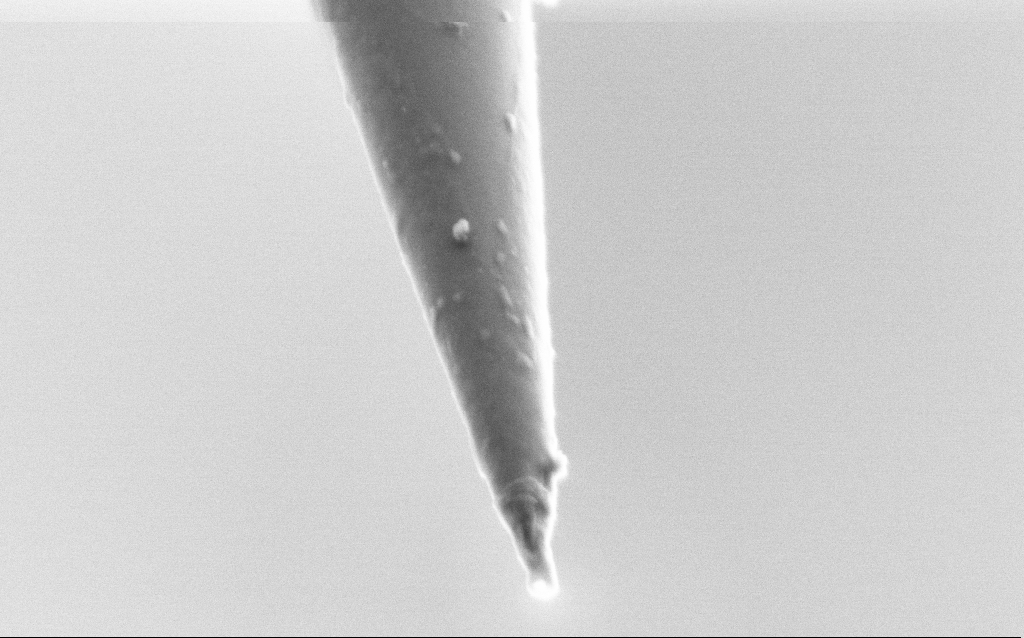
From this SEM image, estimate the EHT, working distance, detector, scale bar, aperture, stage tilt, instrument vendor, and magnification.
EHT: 1.5 kV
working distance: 6 mm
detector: SE2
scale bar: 200 nm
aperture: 30 µm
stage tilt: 45°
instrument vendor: Zeiss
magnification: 100 K X